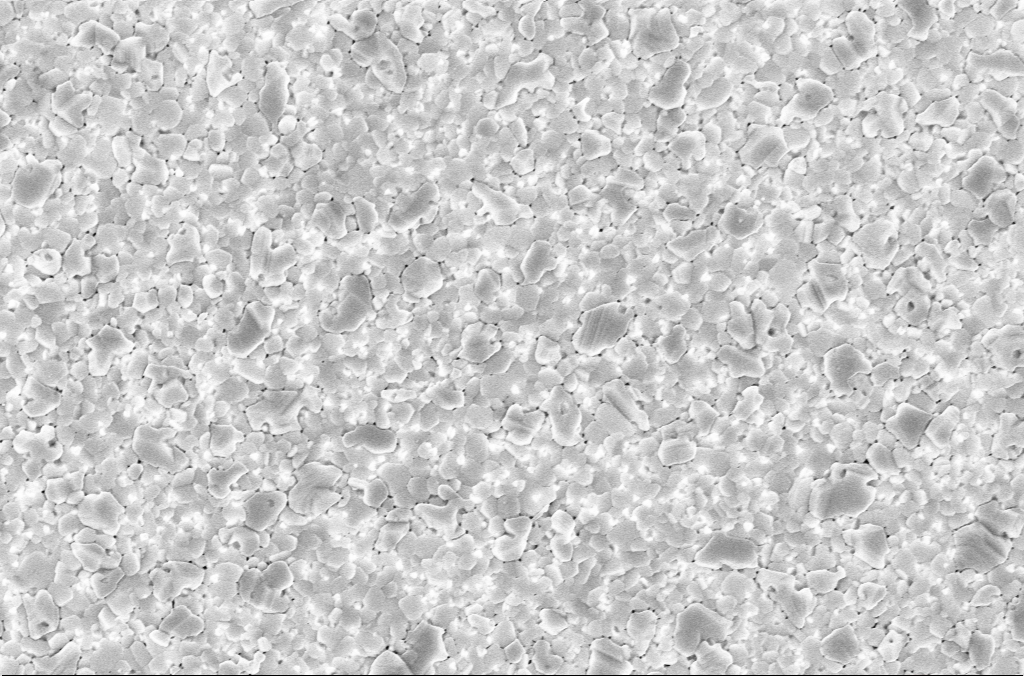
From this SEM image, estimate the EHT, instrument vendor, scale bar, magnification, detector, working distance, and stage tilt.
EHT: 5 kV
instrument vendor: Zeiss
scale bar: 1000 nm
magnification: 30 K X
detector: InLens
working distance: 3 mm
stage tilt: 0°